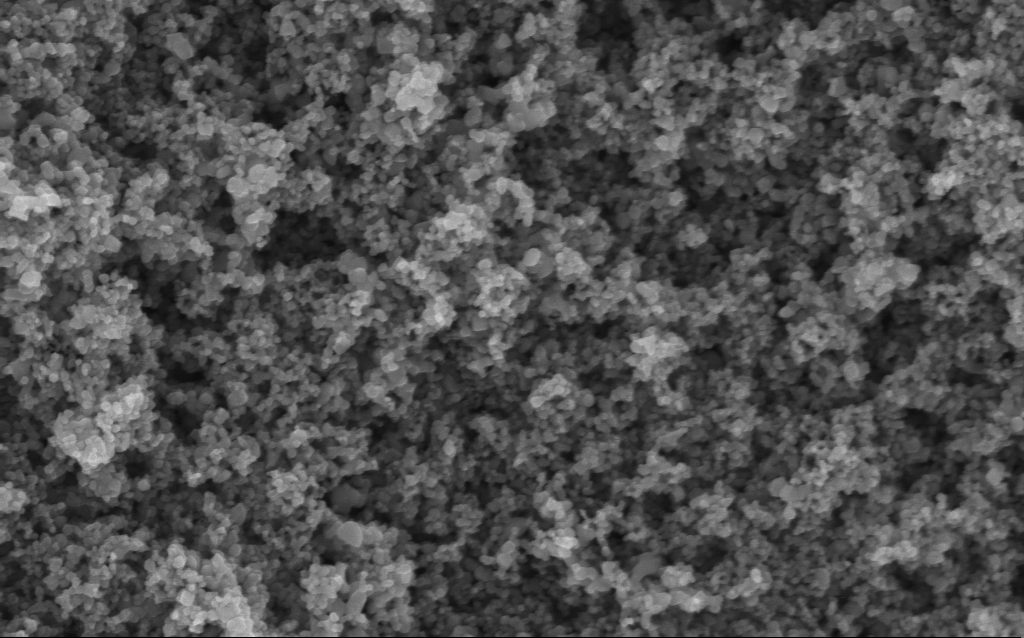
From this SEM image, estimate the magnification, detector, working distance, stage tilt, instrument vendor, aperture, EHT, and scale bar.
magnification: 114.56 K X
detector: InLens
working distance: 4.2 mm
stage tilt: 0°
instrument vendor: Zeiss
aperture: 30 µm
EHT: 5 kV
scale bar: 200 nm